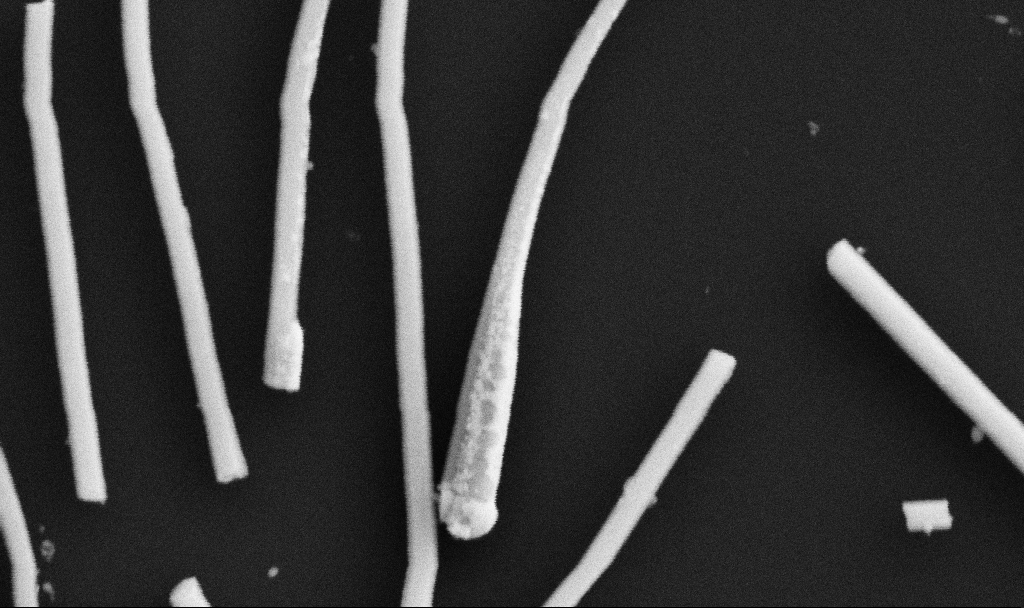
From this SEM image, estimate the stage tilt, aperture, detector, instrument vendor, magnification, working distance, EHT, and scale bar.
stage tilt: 0°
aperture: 30 µm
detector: SE2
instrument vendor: Zeiss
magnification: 105.78 K X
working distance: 10.7 mm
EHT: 5 kV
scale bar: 200 nm